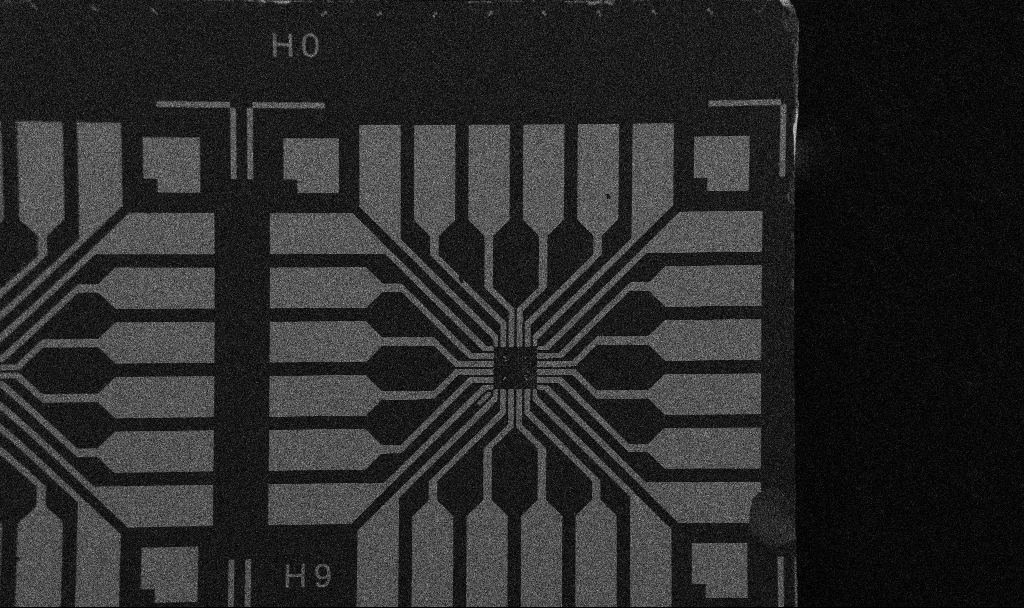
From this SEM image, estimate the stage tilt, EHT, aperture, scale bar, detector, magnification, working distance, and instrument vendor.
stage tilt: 0°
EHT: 5 kV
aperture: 30 µm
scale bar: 200000 nm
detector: SE2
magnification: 0.1 K X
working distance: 10.7 mm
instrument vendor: Zeiss